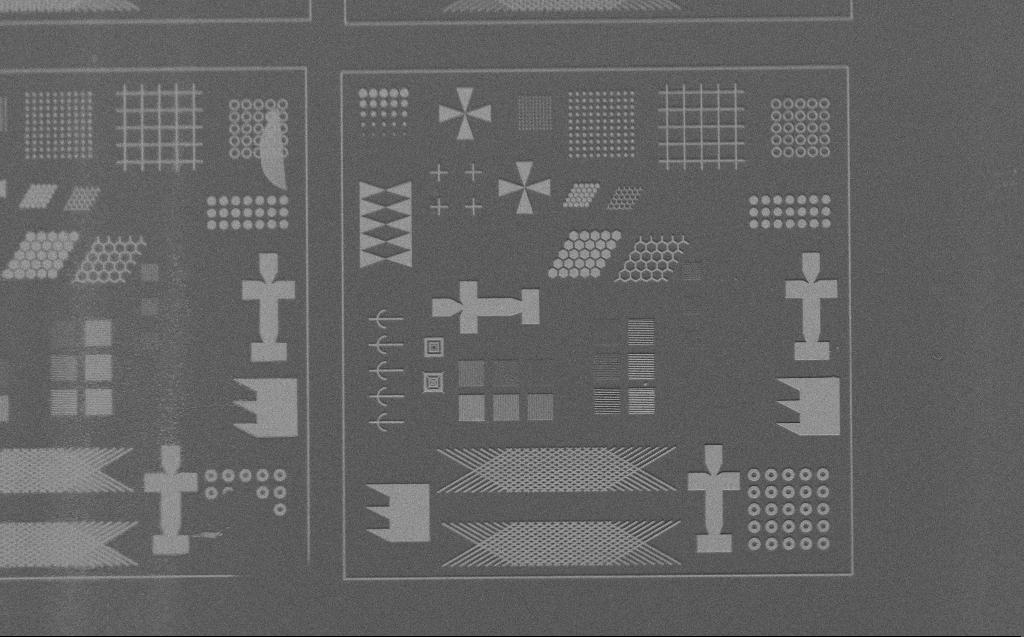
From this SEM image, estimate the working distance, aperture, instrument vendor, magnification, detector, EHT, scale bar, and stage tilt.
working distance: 6 mm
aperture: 30 µm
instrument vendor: Zeiss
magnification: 0.633 K X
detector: SE2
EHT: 3 kV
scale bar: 100000 nm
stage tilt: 45°